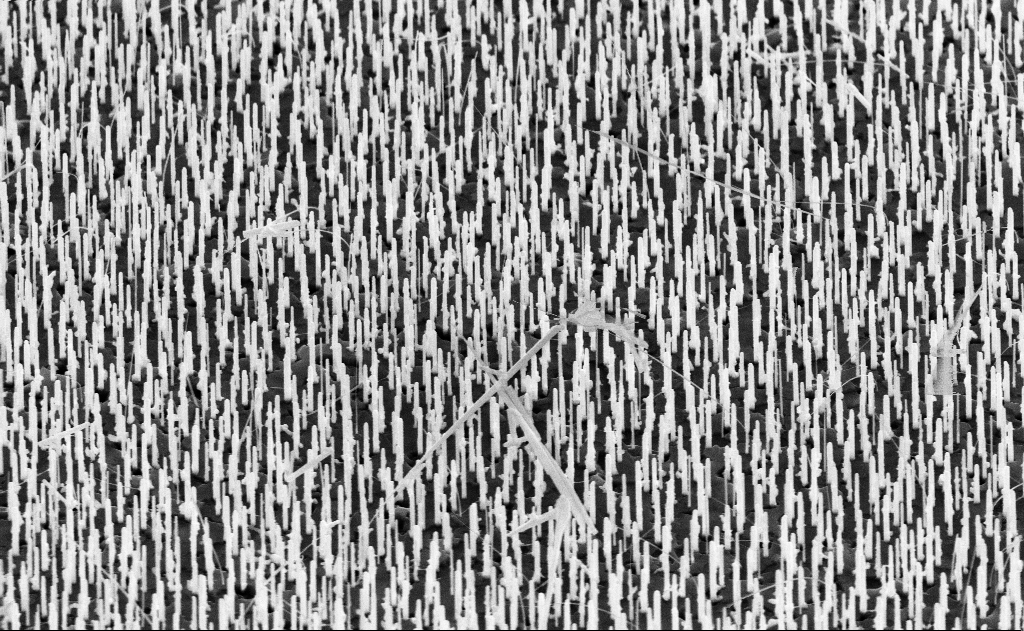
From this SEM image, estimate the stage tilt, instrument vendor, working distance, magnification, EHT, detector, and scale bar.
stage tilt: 45°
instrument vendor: Zeiss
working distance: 14 mm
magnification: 20 K X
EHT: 10 kV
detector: SE2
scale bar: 1000 nm